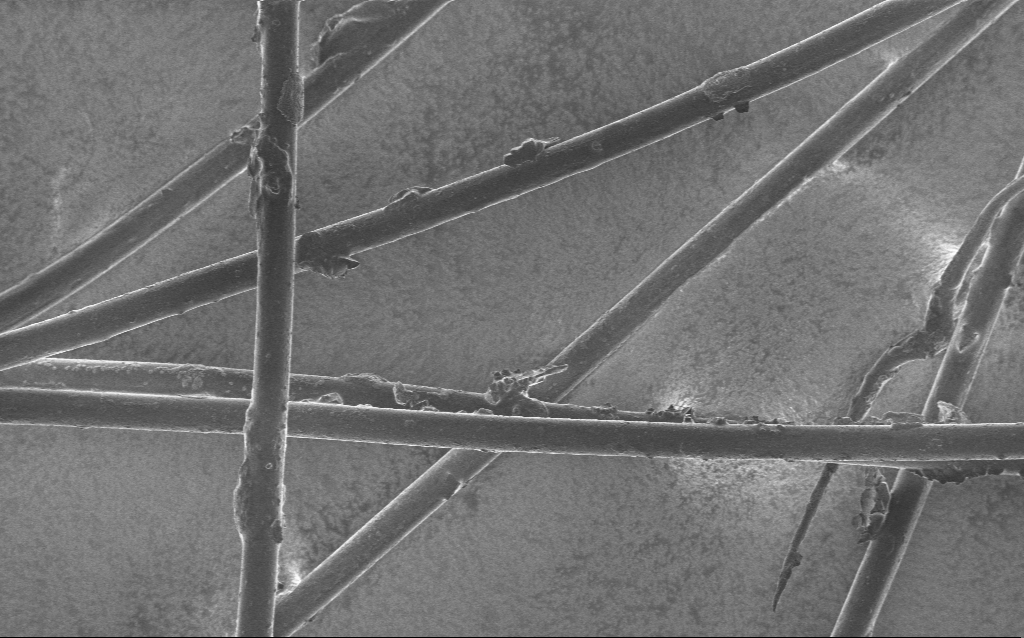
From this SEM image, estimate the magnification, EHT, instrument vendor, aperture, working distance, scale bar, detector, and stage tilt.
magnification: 0.567 K X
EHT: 1 kV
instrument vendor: Zeiss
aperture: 30 µm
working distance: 5 mm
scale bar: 100000 nm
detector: InLens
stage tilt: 0°